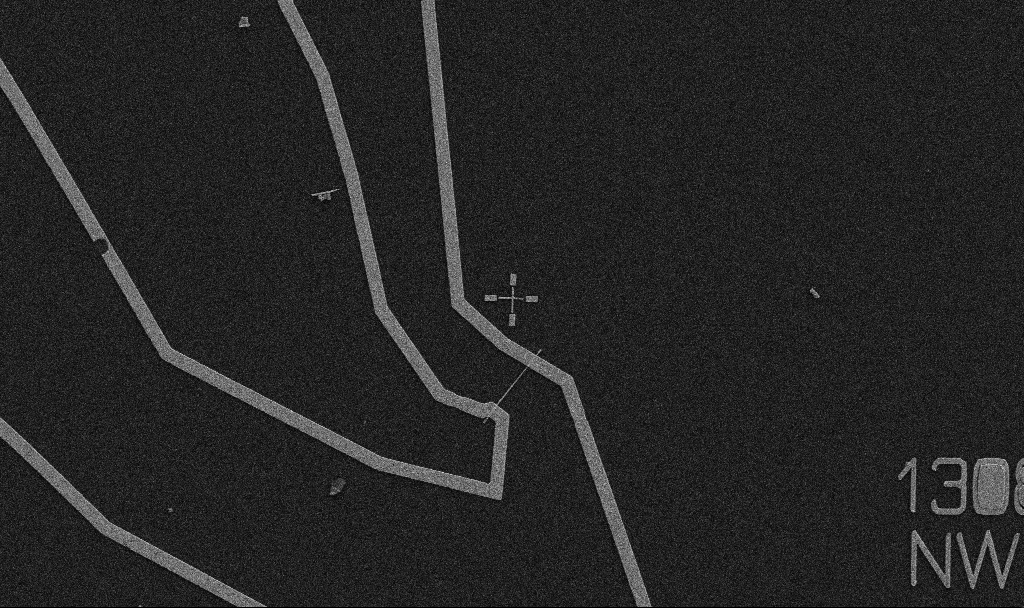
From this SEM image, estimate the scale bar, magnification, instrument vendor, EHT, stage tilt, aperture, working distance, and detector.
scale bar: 10000 nm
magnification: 5 K X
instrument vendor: Zeiss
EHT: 5 kV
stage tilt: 0°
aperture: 30 µm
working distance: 10.7 mm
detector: SE2